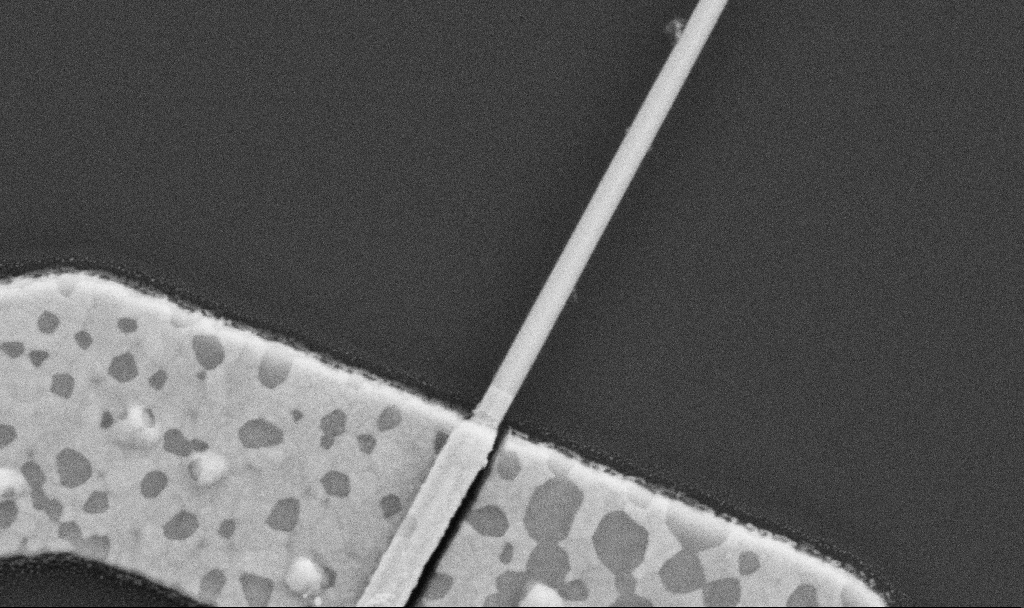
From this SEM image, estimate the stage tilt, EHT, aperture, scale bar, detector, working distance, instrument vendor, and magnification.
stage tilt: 0°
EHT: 5 kV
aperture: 30 µm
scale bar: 200 nm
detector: SE2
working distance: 8.7 mm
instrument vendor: Zeiss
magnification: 100 K X